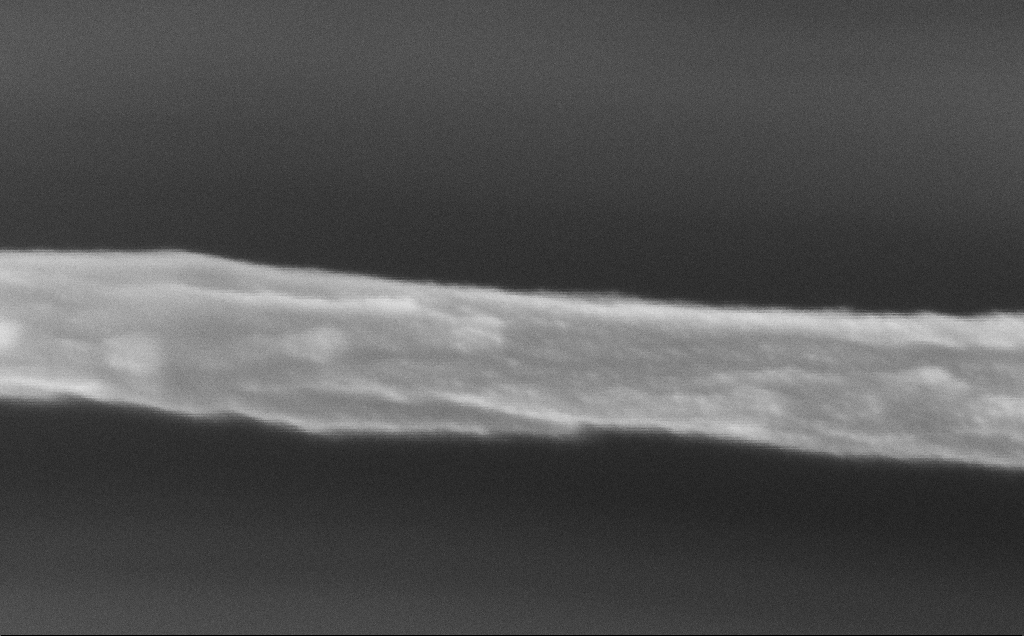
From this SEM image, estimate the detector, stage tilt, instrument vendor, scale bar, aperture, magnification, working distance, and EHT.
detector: InLens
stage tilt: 0°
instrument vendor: Zeiss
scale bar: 100 nm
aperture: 30 µm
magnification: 455.02 K X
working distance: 12 mm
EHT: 5 kV